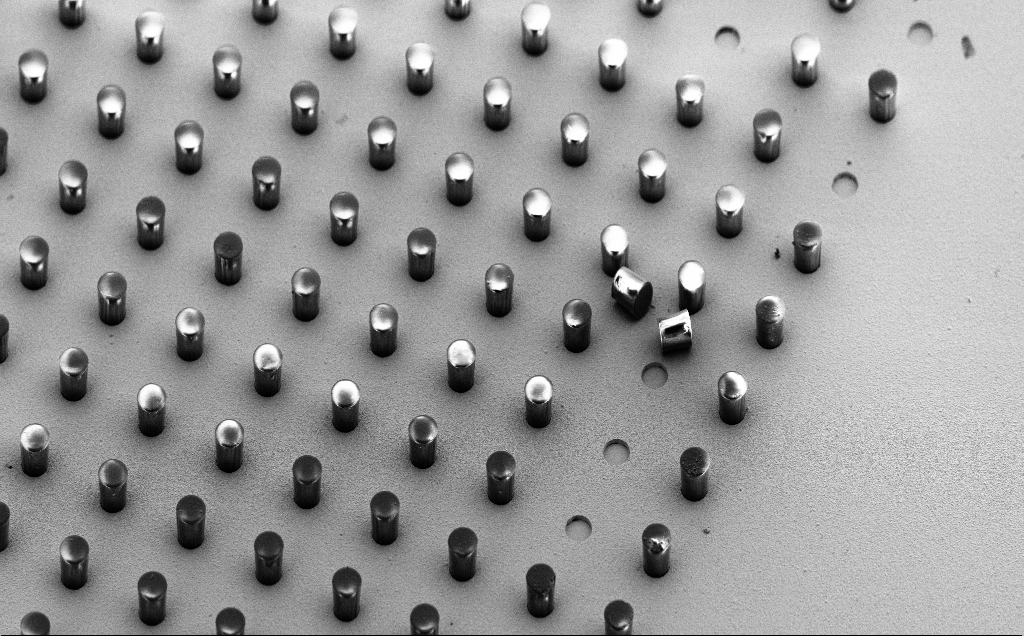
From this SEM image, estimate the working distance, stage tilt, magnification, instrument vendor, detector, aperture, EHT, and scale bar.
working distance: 8 mm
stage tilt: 45°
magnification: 0.534 K X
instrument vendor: Zeiss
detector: SE2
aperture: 30 µm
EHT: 5 kV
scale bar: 100000 nm